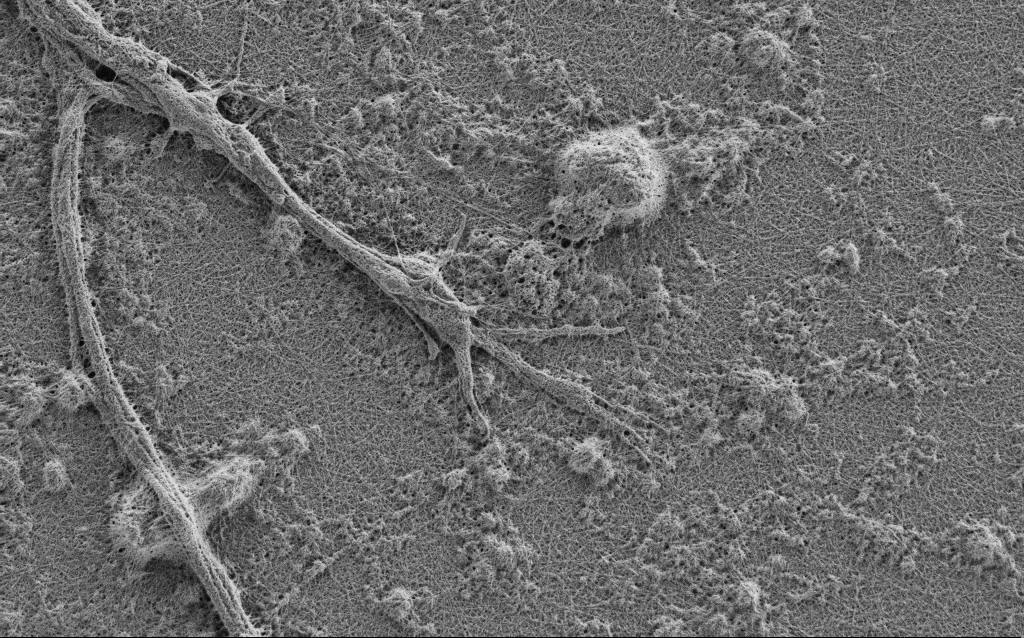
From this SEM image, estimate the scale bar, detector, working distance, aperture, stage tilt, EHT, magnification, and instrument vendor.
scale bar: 2000 nm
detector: SE2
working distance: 4 mm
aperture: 30 µm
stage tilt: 0°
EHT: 0.9 kV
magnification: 10 K X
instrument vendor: Zeiss